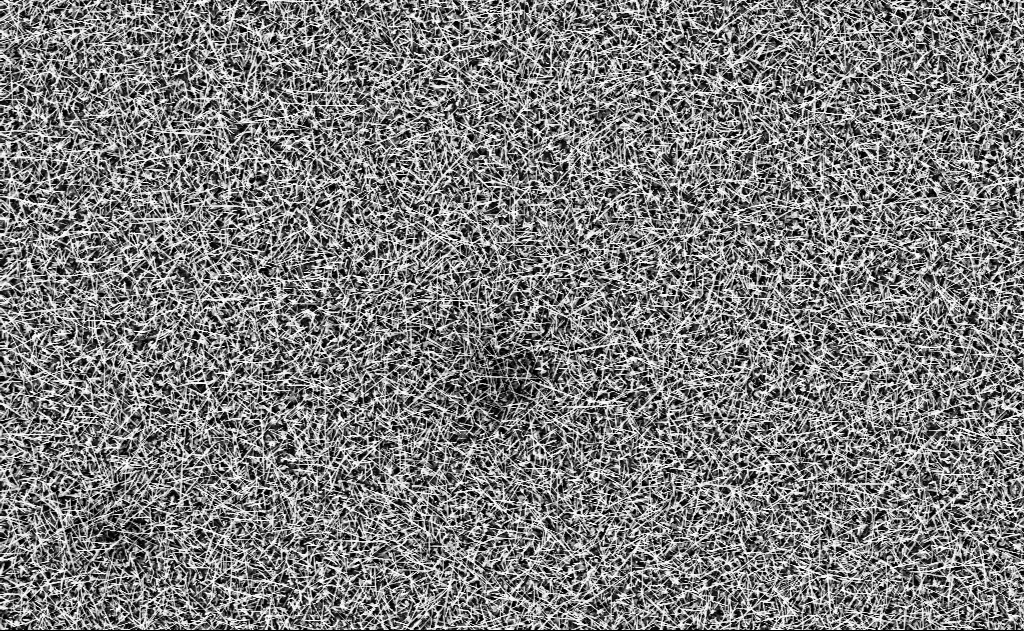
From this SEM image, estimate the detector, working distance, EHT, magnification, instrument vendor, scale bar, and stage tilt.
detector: InLens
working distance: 15 mm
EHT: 10 kV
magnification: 10 K X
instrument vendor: Zeiss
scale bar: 2000 nm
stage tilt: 0°